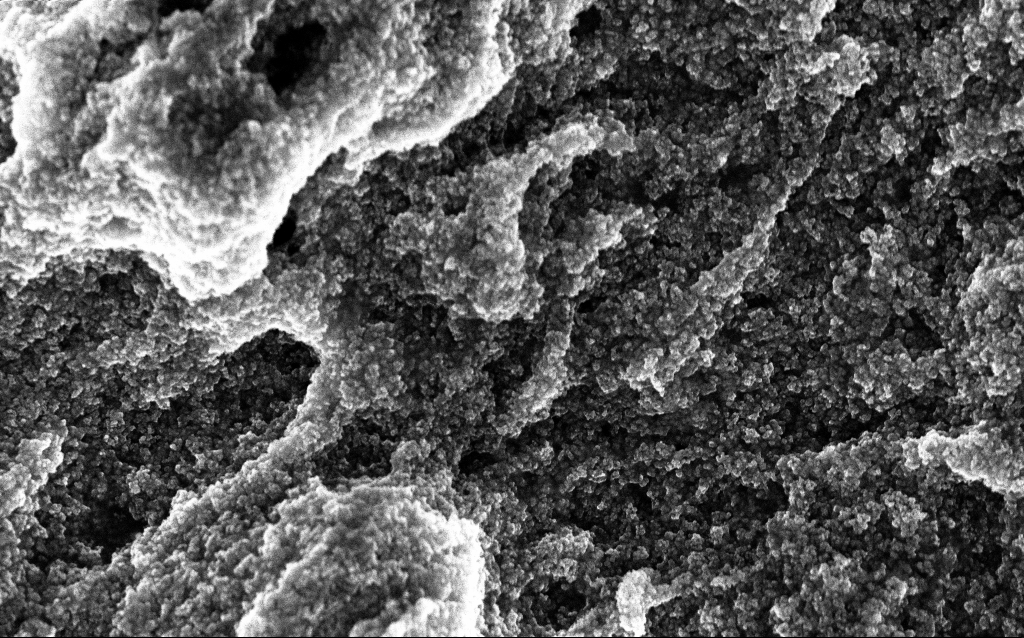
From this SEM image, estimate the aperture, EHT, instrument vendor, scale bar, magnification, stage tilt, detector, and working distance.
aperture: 30 µm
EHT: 10 kV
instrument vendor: Zeiss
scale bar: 1000 nm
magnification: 37.88 K X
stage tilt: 0°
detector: InLens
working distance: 2.6 mm